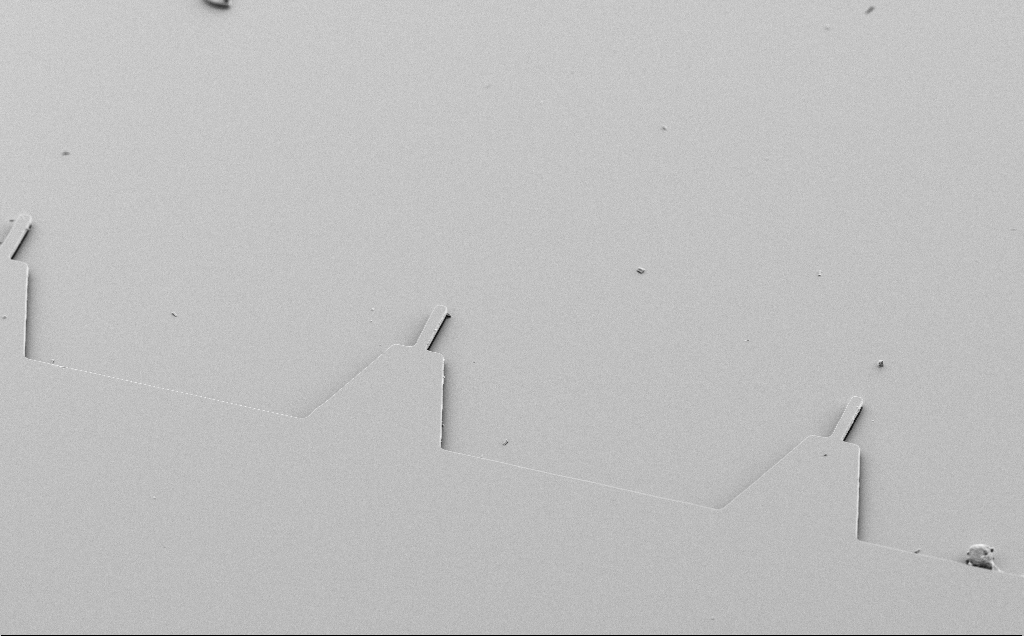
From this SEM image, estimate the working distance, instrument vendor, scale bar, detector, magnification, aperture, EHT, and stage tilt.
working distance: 10 mm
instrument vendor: Zeiss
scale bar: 20000 nm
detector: SE2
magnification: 1.01 K X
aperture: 30 µm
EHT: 5 kV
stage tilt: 50°